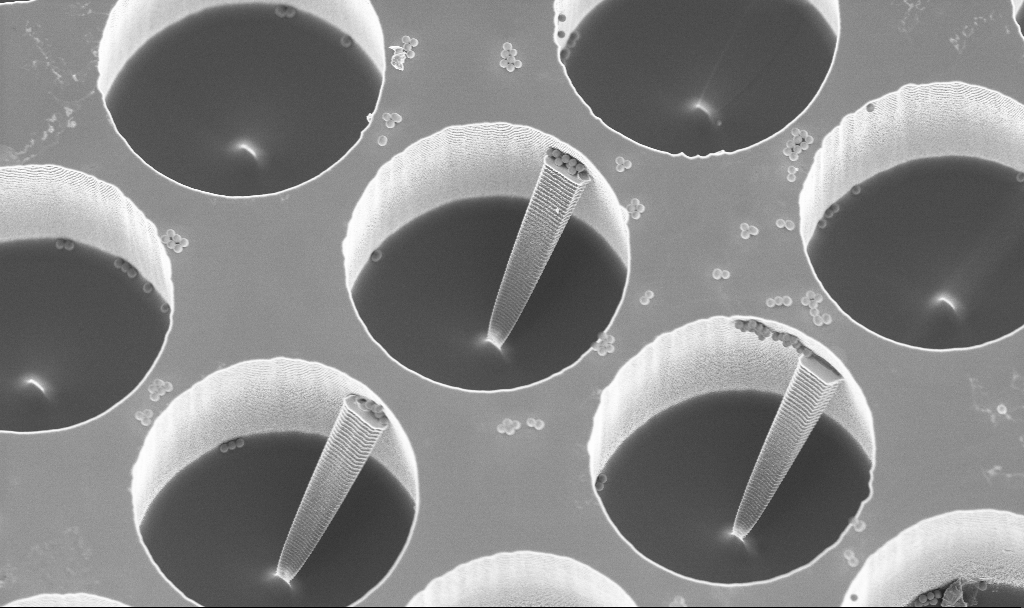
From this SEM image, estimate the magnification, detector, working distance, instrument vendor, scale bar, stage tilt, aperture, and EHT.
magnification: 5.19 K X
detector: InLens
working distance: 3 mm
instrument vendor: Zeiss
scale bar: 10000 nm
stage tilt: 20°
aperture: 30 µm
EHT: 3 kV